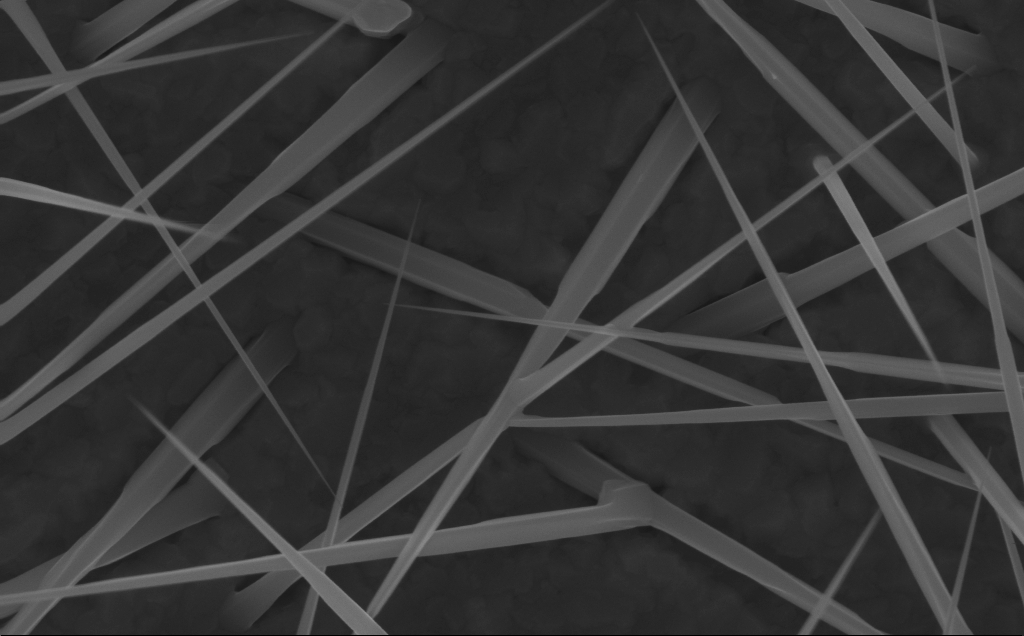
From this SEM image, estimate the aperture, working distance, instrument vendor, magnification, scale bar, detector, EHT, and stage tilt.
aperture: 30 µm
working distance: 6 mm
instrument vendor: Zeiss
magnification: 80 K X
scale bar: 200 nm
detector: InLens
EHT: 10 kV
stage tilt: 0°